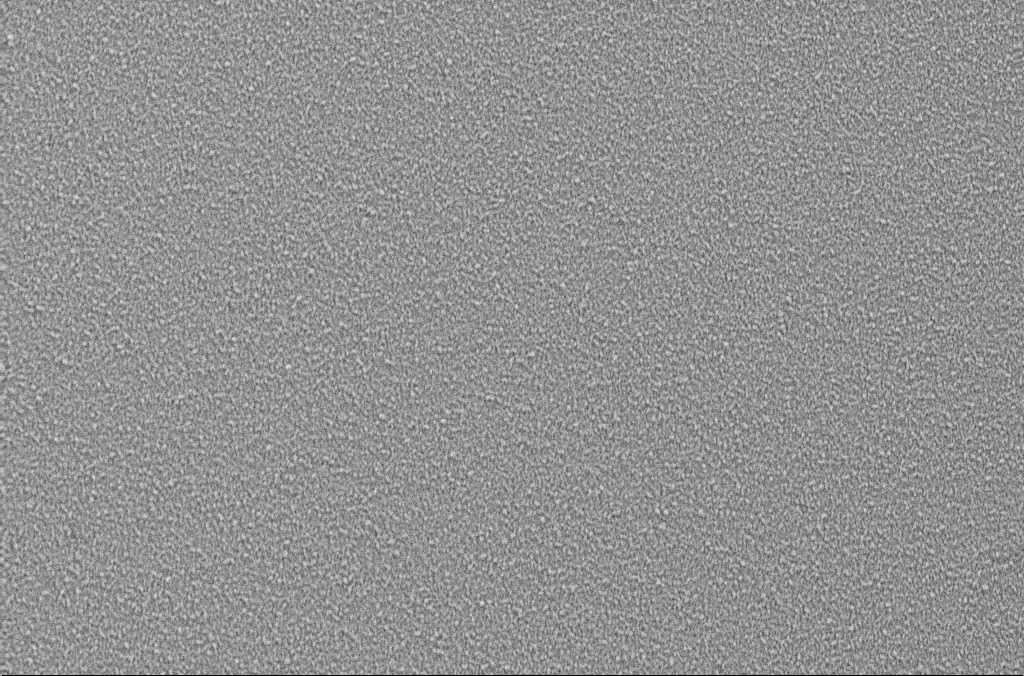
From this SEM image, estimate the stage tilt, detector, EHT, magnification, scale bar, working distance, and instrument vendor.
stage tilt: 0°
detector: SE2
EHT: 5 kV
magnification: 5 K X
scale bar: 10000 nm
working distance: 1.9 mm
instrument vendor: Zeiss